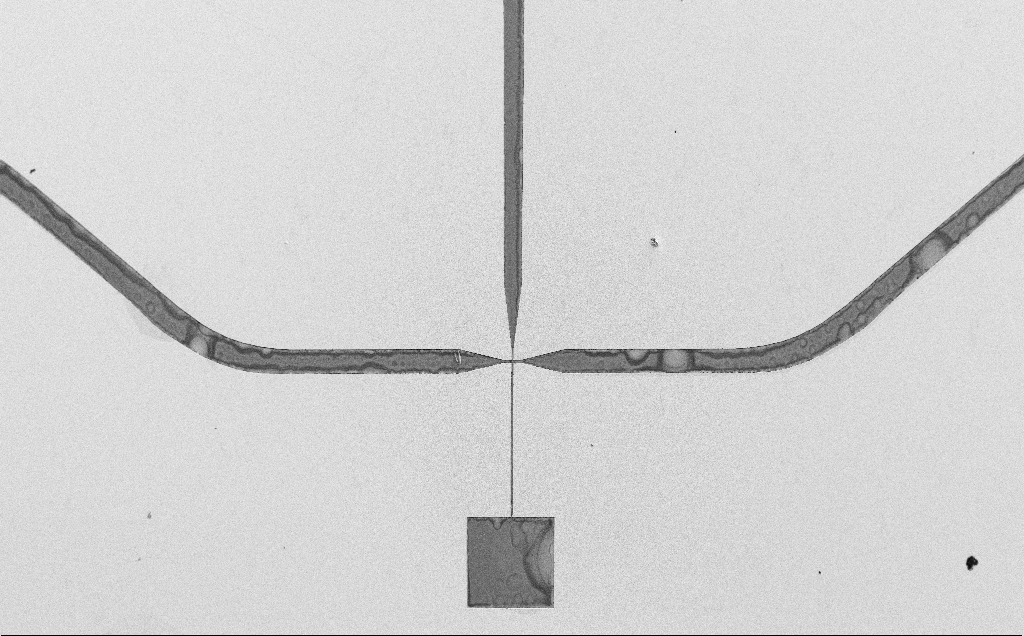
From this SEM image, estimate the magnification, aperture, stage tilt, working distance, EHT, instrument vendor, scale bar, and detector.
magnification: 0.082 K X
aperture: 30 µm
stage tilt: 0°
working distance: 14 mm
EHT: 10 kV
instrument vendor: Zeiss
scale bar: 200000 nm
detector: SE2